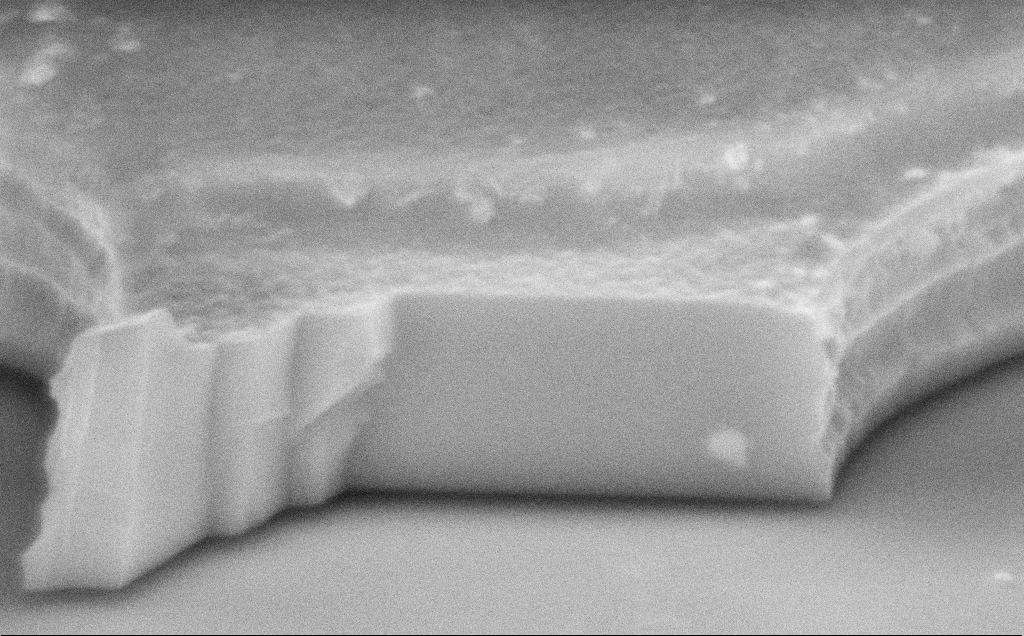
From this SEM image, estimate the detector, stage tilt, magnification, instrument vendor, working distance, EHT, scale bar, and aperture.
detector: InLens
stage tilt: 70°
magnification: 48.51 K X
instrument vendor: Zeiss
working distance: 12 mm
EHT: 8 kV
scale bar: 1000 nm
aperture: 30 µm